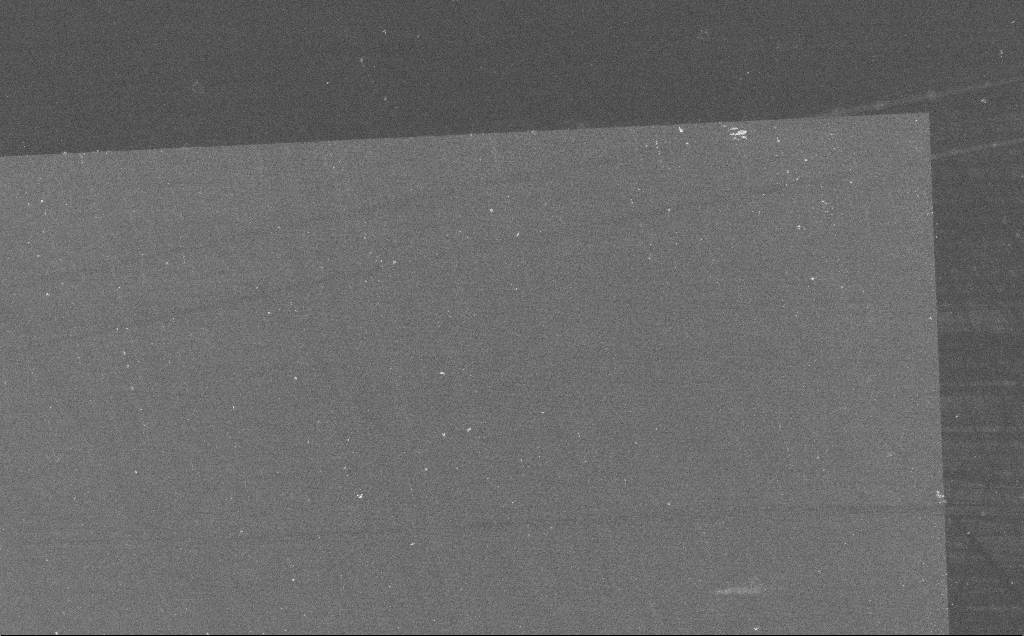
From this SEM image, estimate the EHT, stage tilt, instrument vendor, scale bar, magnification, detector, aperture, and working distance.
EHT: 10 kV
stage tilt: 0°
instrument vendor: Zeiss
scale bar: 100000 nm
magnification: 0.493 K X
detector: InLens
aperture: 30 µm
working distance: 9 mm